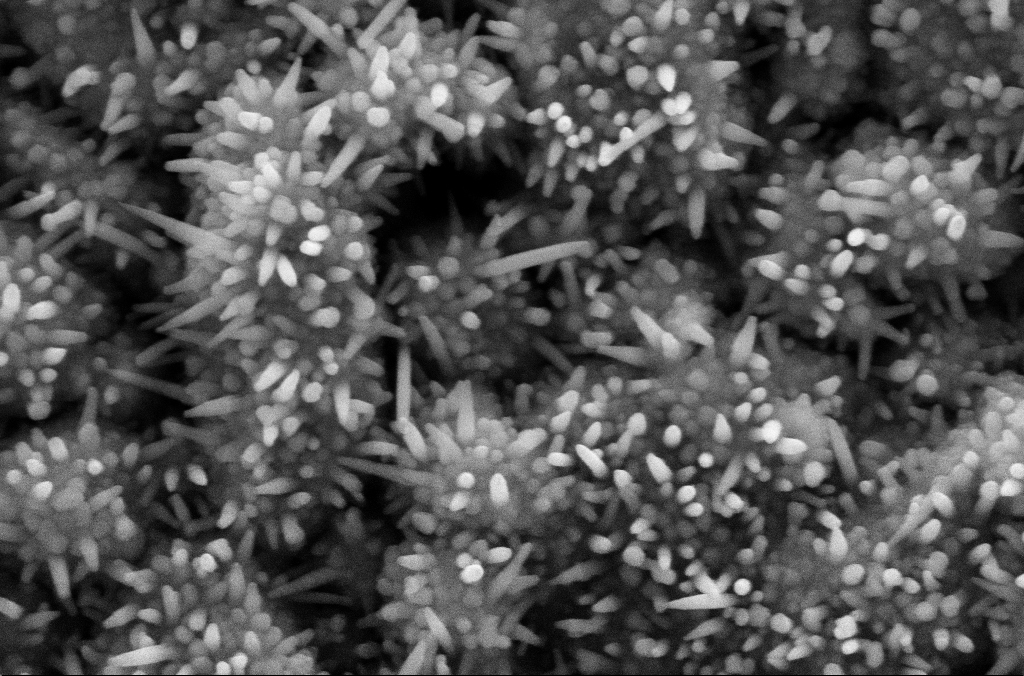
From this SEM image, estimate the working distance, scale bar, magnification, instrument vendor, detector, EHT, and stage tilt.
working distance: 5.2 mm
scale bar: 200 nm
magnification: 96.07 K X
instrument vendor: Zeiss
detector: SE2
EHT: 10 kV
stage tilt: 0.1°